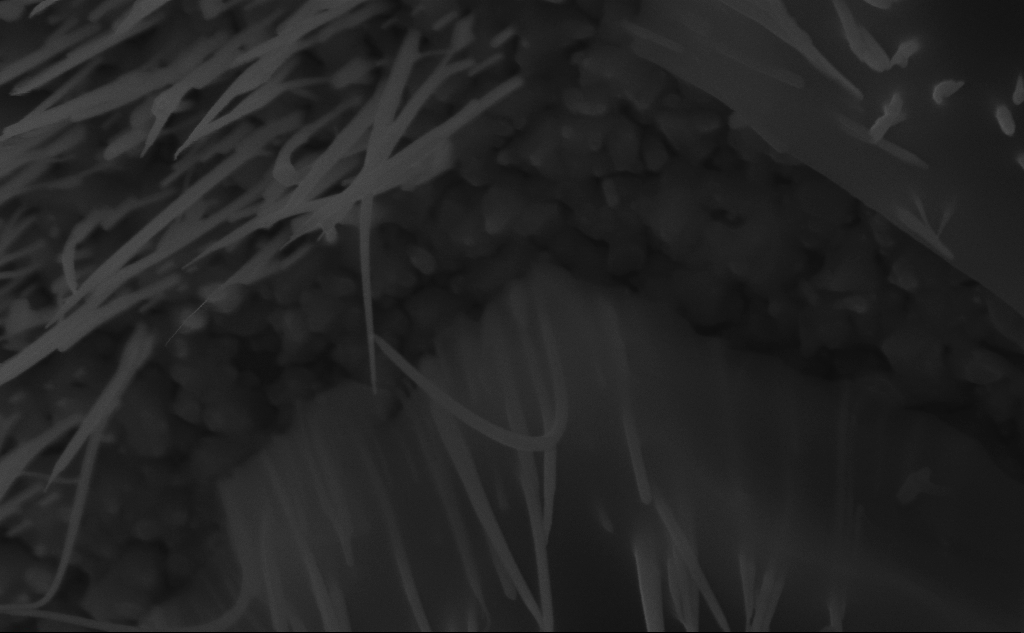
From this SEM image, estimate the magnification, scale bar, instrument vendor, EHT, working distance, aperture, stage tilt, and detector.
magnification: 98.96 K X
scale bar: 200 nm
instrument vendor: Zeiss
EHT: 10 kV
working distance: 6 mm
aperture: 30 µm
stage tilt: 45°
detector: InLens